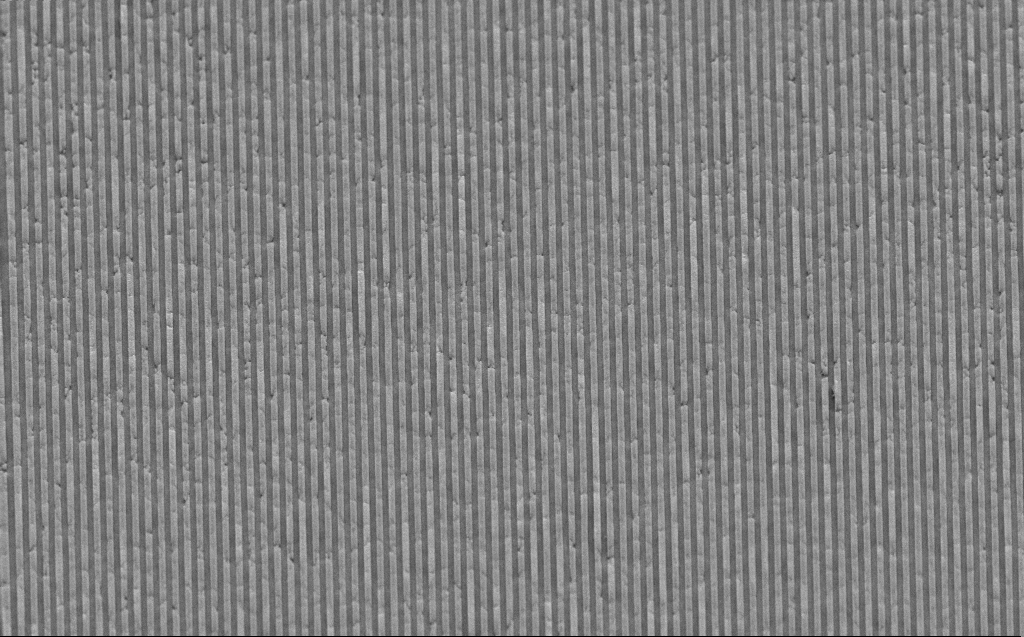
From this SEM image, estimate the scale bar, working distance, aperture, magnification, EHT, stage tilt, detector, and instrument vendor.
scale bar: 2000 nm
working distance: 8 mm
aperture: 30 µm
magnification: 9.51 K X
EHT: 10 kV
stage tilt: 45°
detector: SE2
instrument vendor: Zeiss